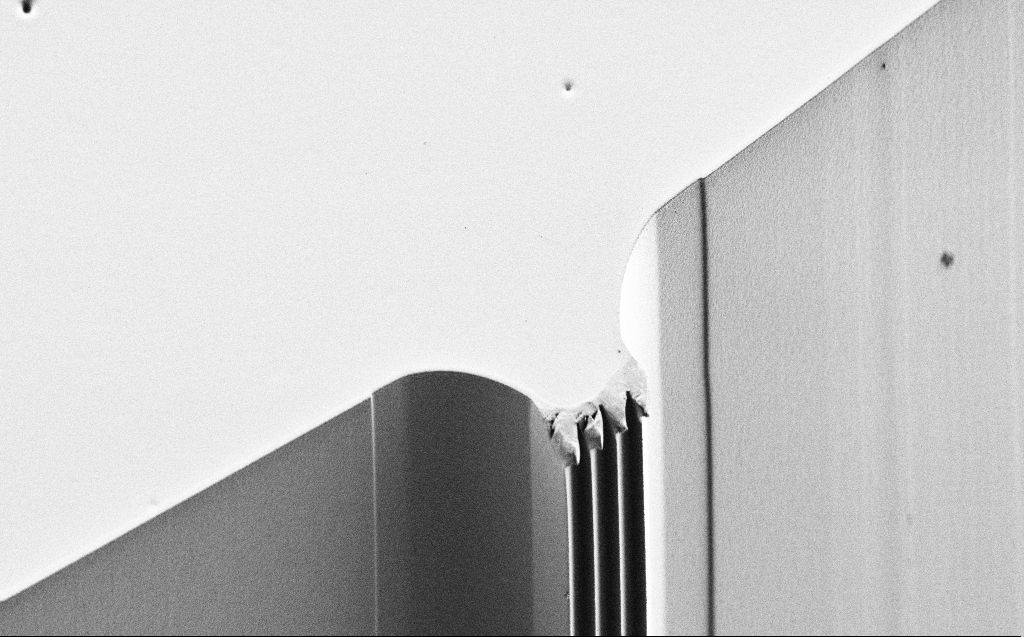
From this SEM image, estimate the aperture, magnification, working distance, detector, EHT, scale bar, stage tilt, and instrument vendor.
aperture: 30 µm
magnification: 0.959 K X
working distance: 5 mm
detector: SE2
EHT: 1 kV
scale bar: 20000 nm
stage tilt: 44.6°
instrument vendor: Zeiss